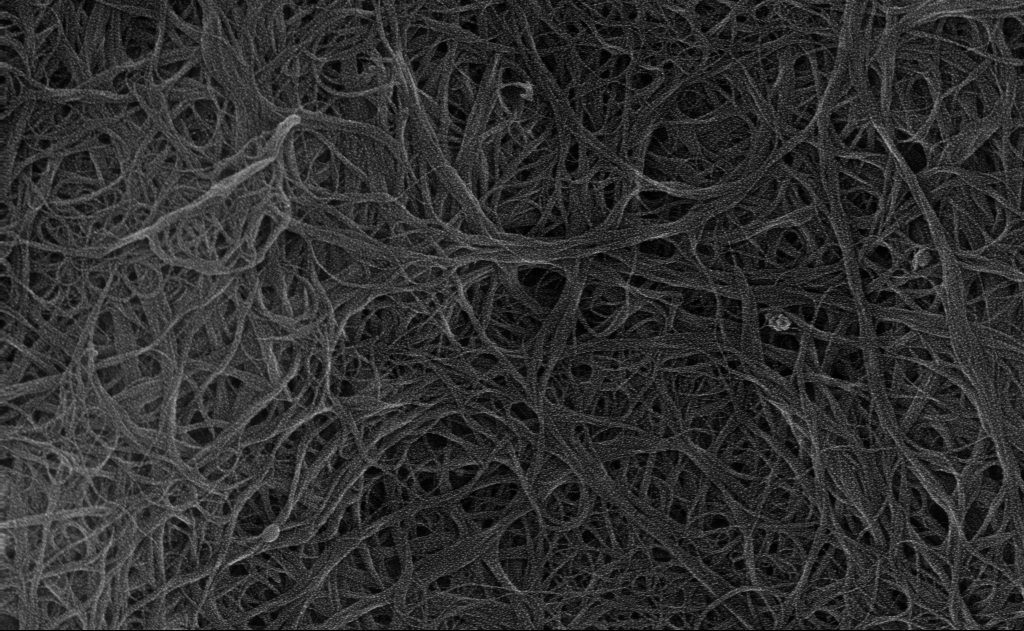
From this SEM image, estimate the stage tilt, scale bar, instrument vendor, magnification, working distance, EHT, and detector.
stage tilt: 0°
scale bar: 200 nm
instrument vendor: Zeiss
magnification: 86.17 K X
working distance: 3 mm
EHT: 10 kV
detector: InLens